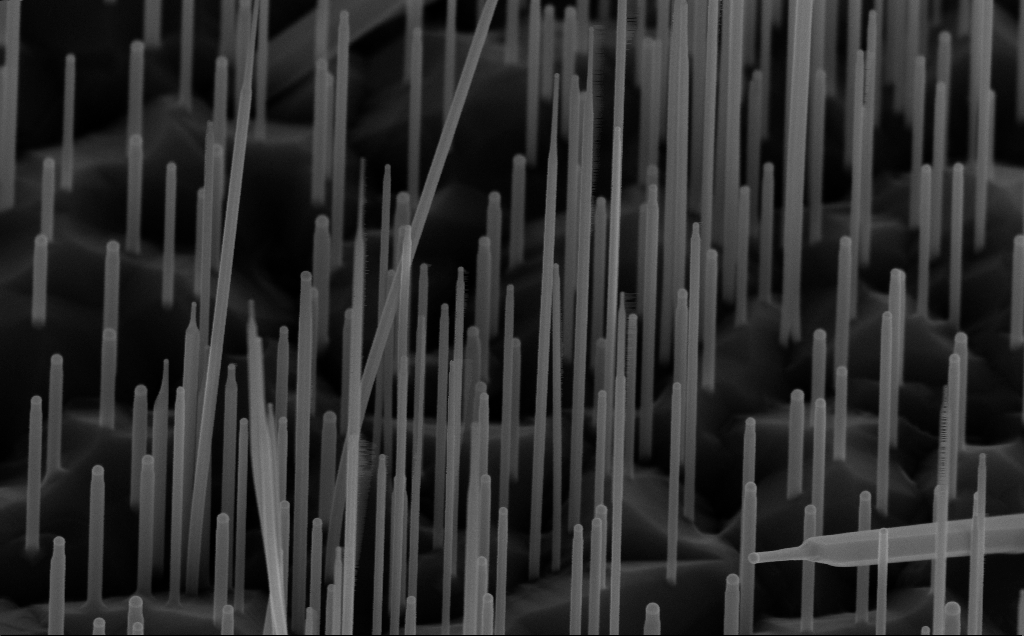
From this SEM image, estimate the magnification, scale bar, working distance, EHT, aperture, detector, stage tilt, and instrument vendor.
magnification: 80 K X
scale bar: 200 nm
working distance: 7 mm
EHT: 10 kV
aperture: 30 µm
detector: InLens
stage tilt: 45°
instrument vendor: Zeiss